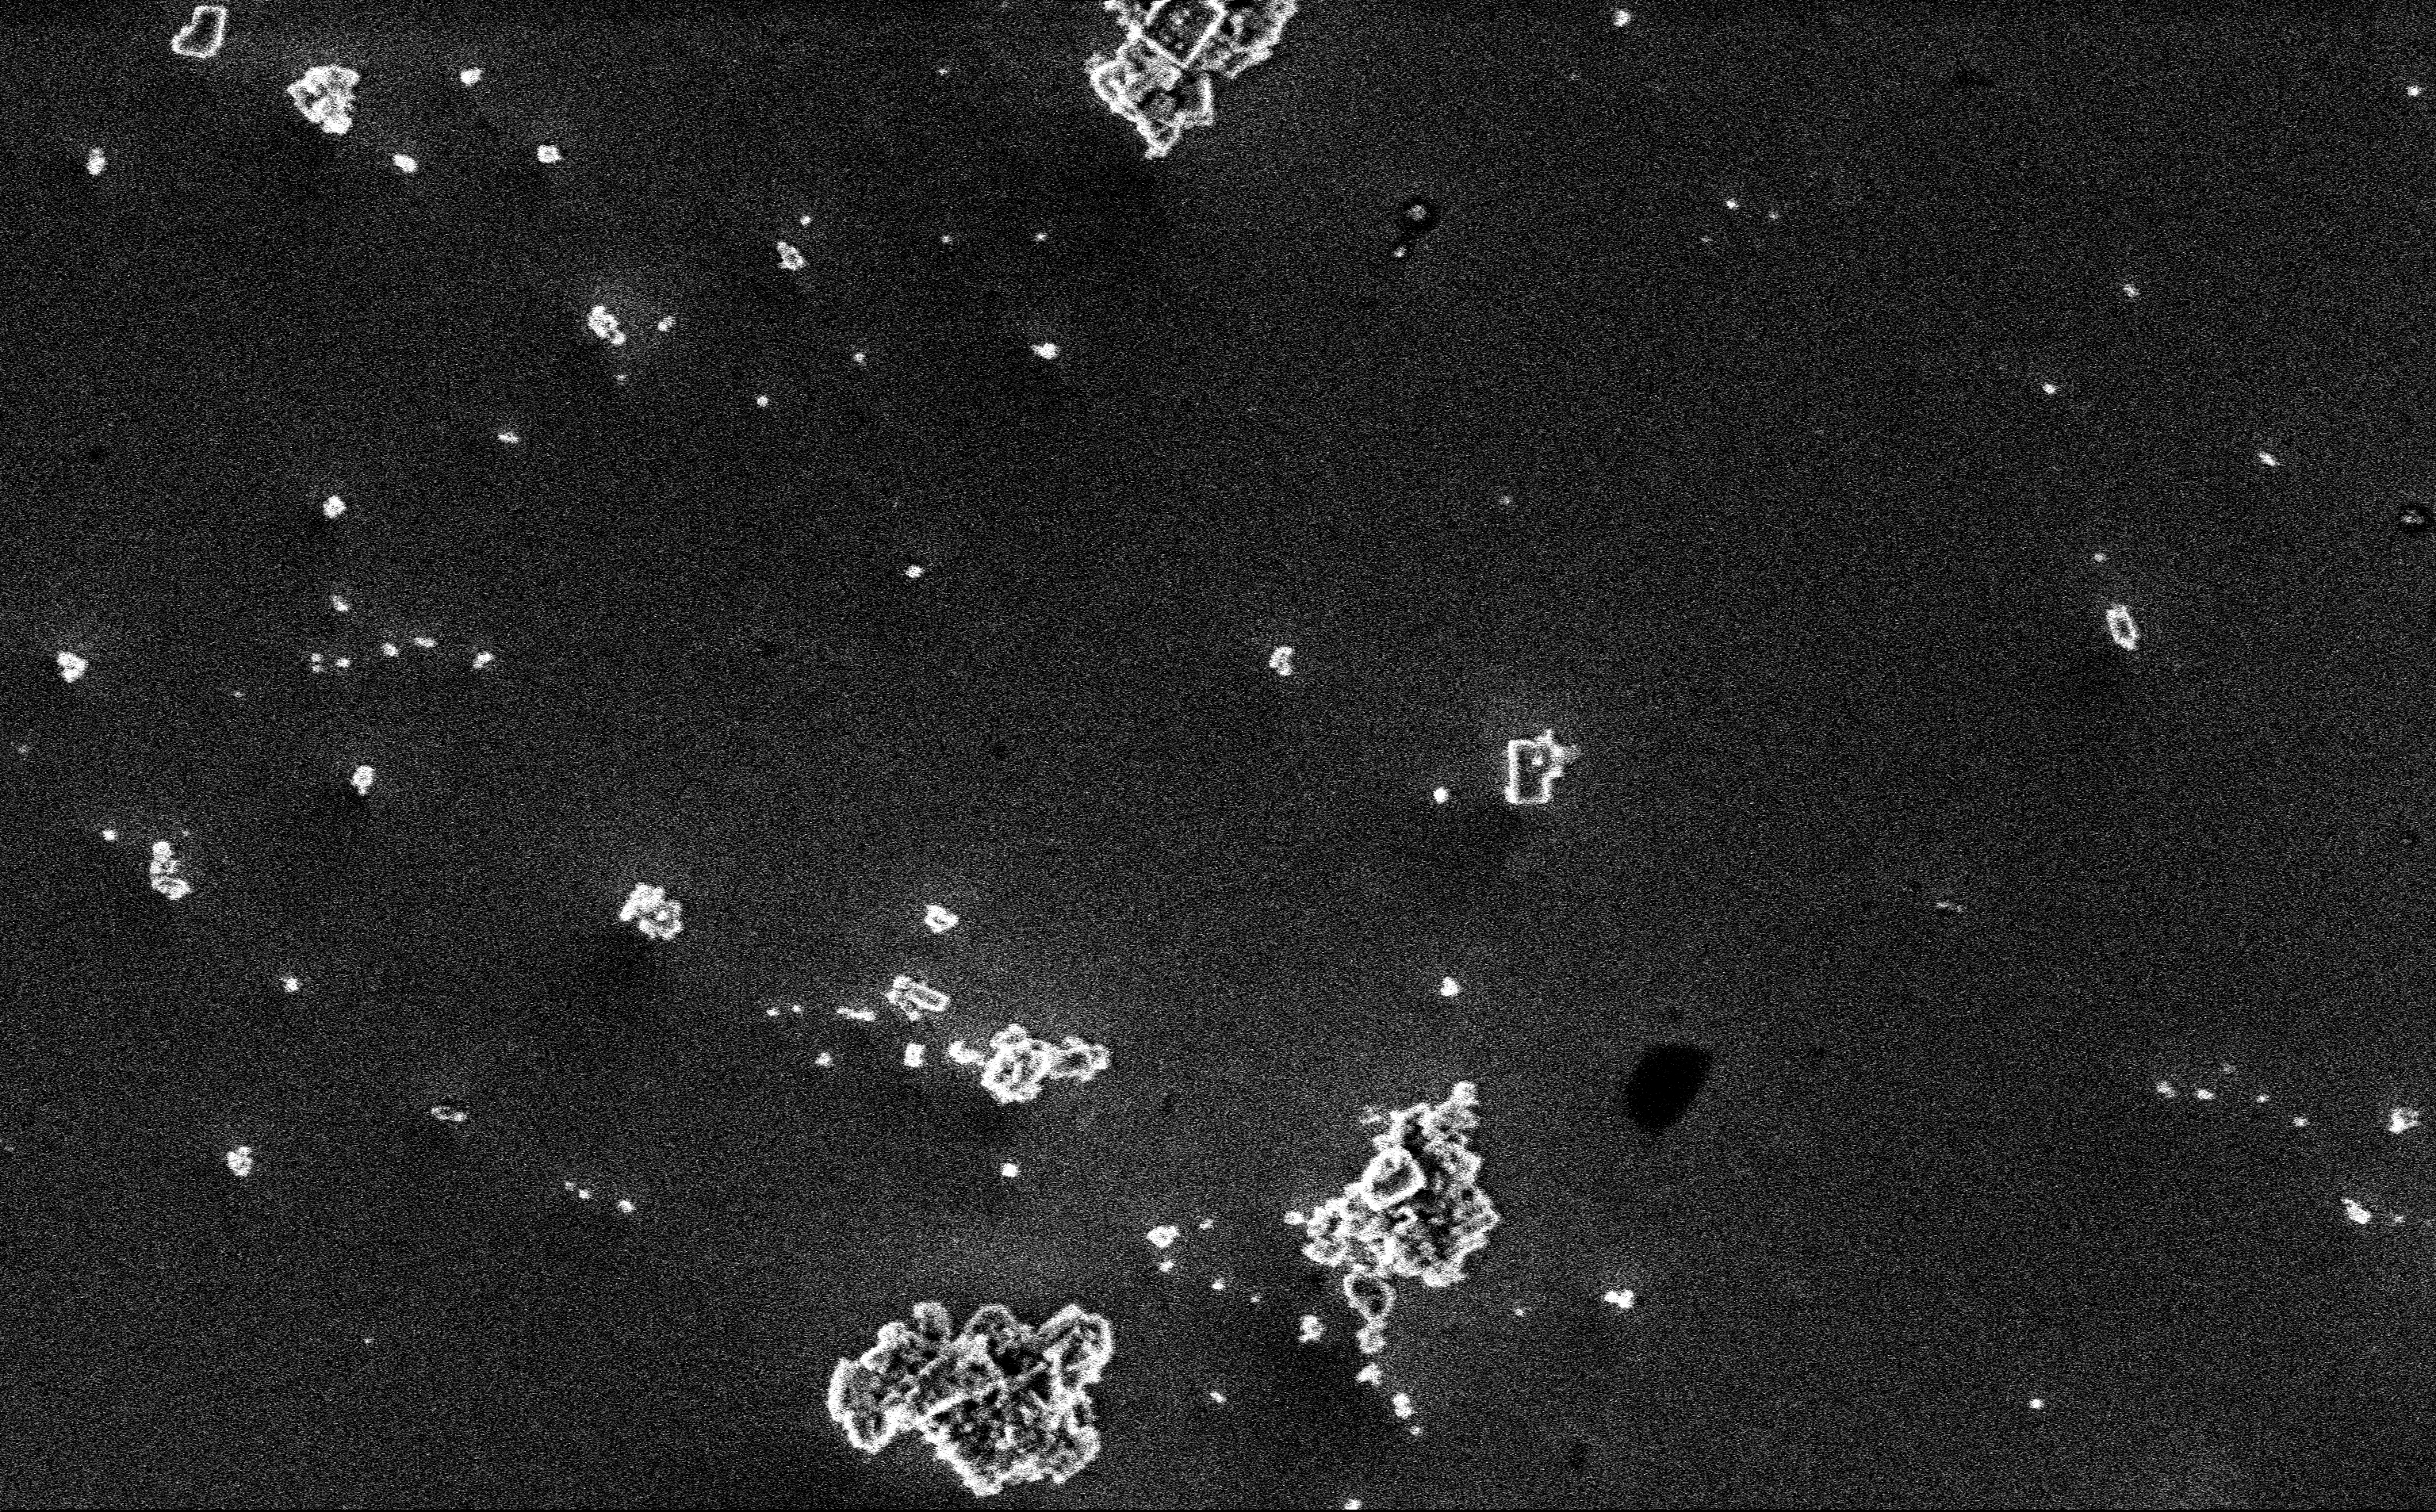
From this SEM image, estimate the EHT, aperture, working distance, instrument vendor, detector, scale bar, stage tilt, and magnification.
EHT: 3 kV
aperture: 30 µm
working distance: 3 mm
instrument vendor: Zeiss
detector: InLens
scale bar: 2000 nm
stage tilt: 0°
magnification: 12.85 K X